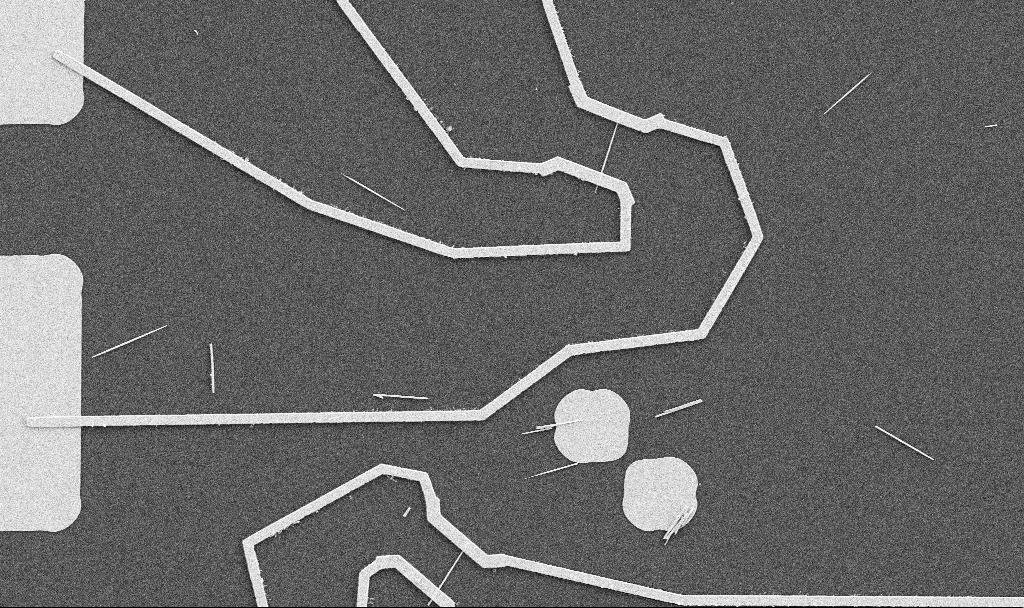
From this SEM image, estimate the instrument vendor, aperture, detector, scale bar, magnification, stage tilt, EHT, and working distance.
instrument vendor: Zeiss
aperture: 30 µm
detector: SE2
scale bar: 10000 nm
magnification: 5 K X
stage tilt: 0°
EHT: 5 kV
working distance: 10.7 mm